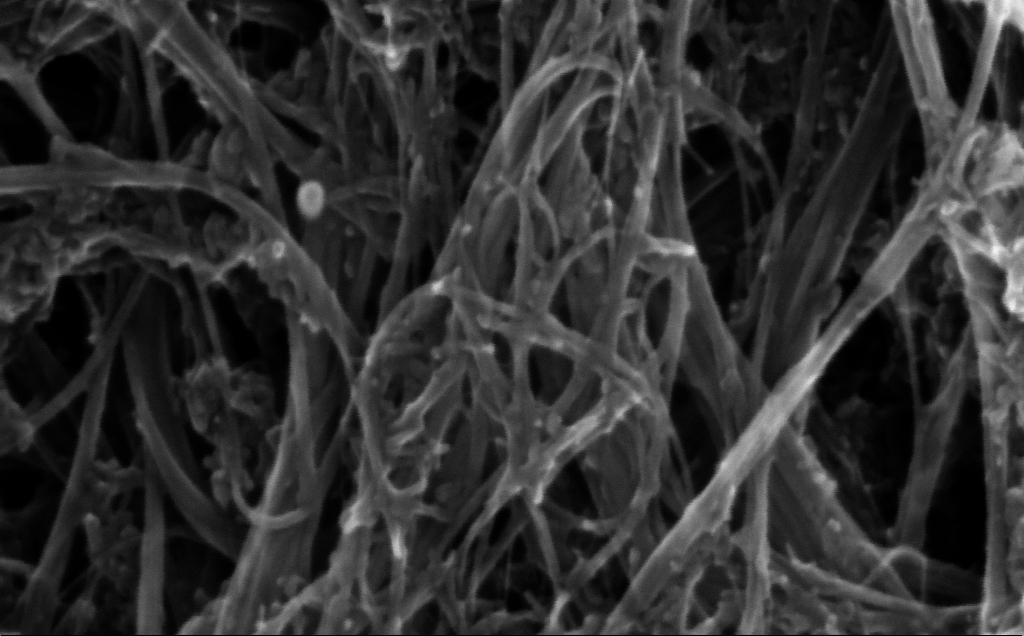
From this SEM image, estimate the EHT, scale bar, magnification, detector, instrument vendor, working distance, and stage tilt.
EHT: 5 kV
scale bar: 100 nm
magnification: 390.27 K X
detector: InLens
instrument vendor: Zeiss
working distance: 4 mm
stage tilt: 0°